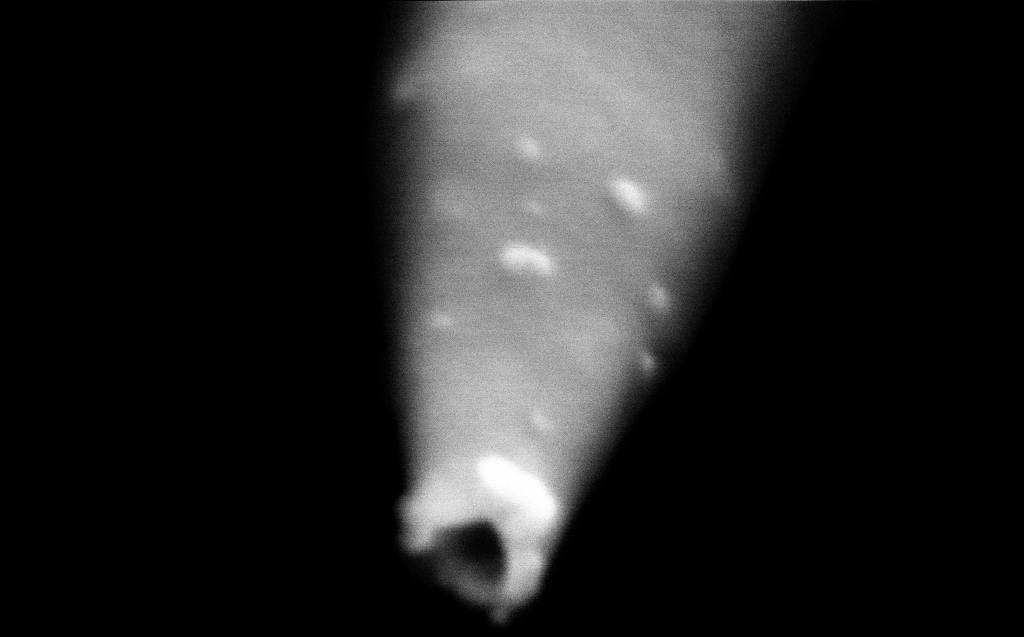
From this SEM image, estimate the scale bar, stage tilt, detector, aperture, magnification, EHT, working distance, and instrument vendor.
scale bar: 100 nm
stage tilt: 45°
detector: InLens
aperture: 30 µm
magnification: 500 K X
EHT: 2 kV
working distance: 4 mm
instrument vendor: Zeiss